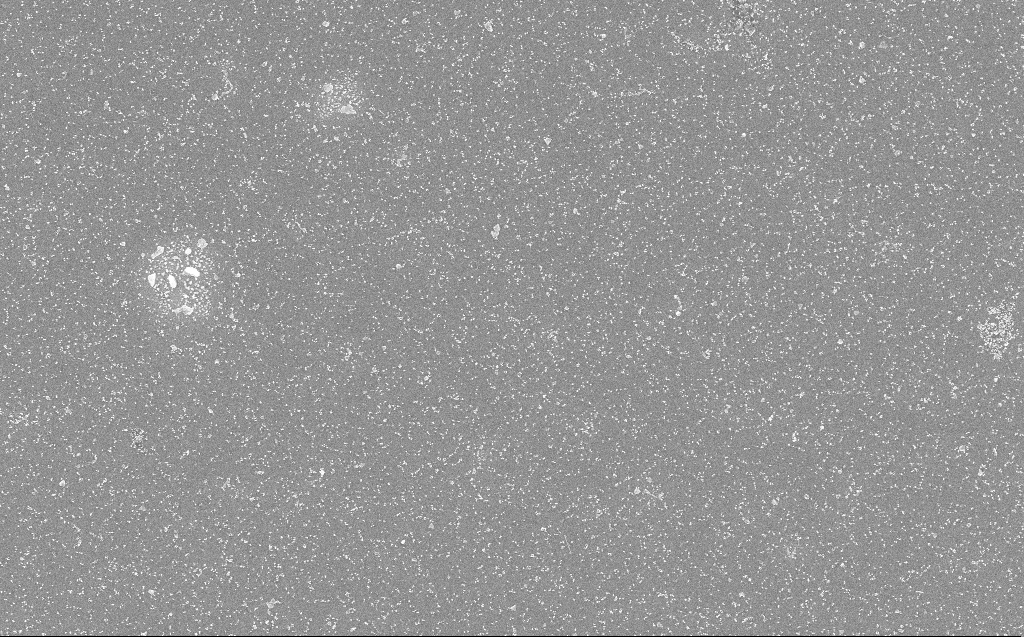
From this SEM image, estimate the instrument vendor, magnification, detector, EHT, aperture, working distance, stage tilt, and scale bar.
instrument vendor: Zeiss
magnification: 10 K X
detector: InLens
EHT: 10 kV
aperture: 30 µm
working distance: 3 mm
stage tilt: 0°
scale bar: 2000 nm